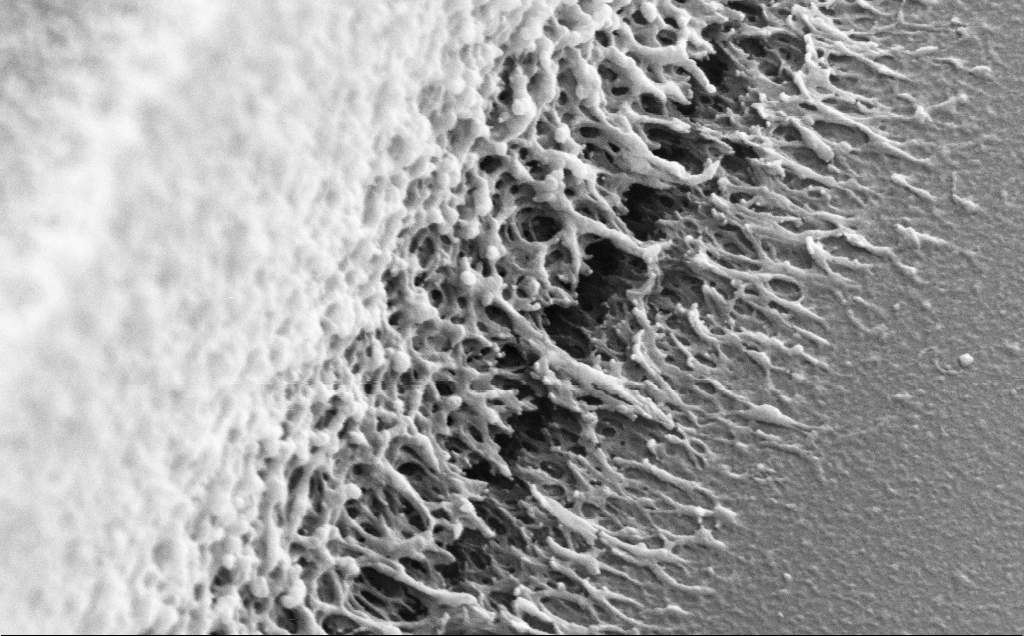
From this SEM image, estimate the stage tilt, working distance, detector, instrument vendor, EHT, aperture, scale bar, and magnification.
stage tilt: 30°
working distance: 10 mm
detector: SE2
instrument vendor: Zeiss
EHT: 5 kV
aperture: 30 µm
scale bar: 200 nm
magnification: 82.73 K X